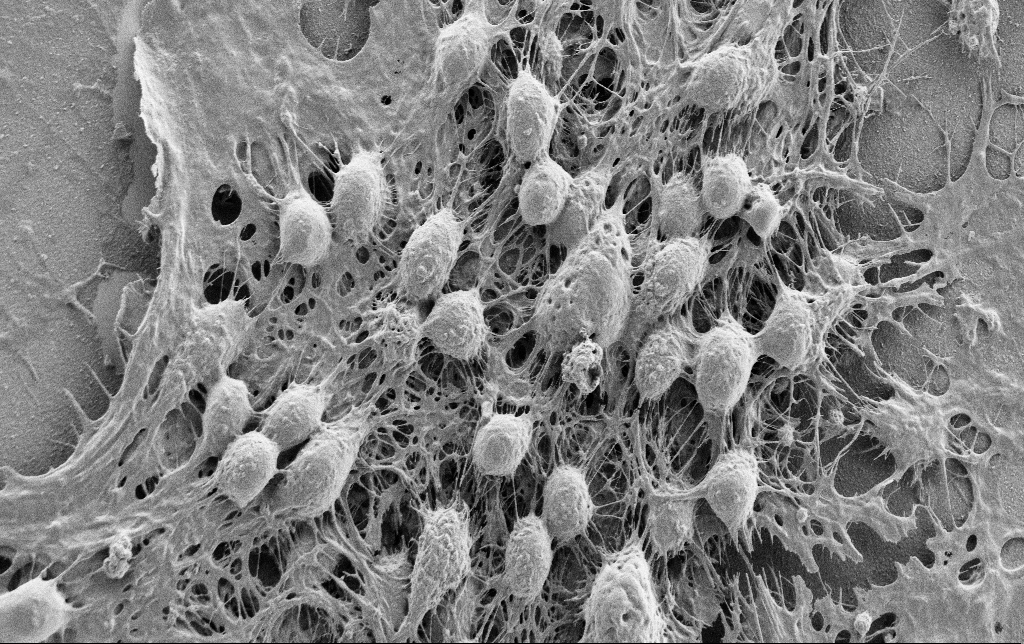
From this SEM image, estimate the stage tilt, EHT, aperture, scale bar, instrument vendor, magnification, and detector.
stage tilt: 0°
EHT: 0.9 kV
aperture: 30 µm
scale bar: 10000 nm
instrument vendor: Zeiss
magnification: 4 K X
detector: SE2